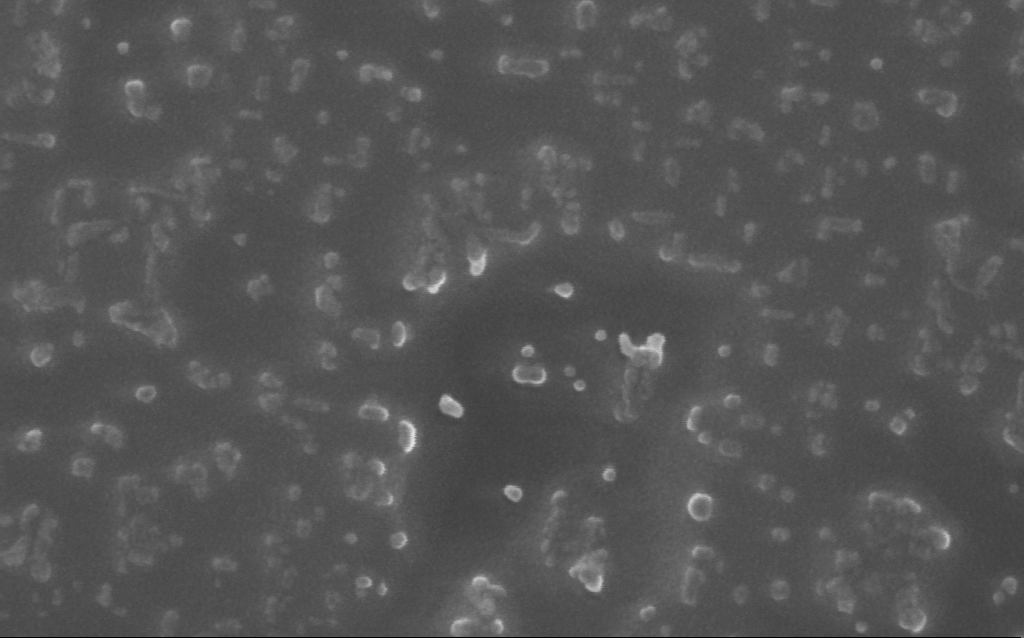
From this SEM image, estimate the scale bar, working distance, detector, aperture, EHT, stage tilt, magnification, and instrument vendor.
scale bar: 200 nm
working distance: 5 mm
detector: InLens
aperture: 30 µm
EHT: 10 kV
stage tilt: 0°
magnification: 318.66 K X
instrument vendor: Zeiss